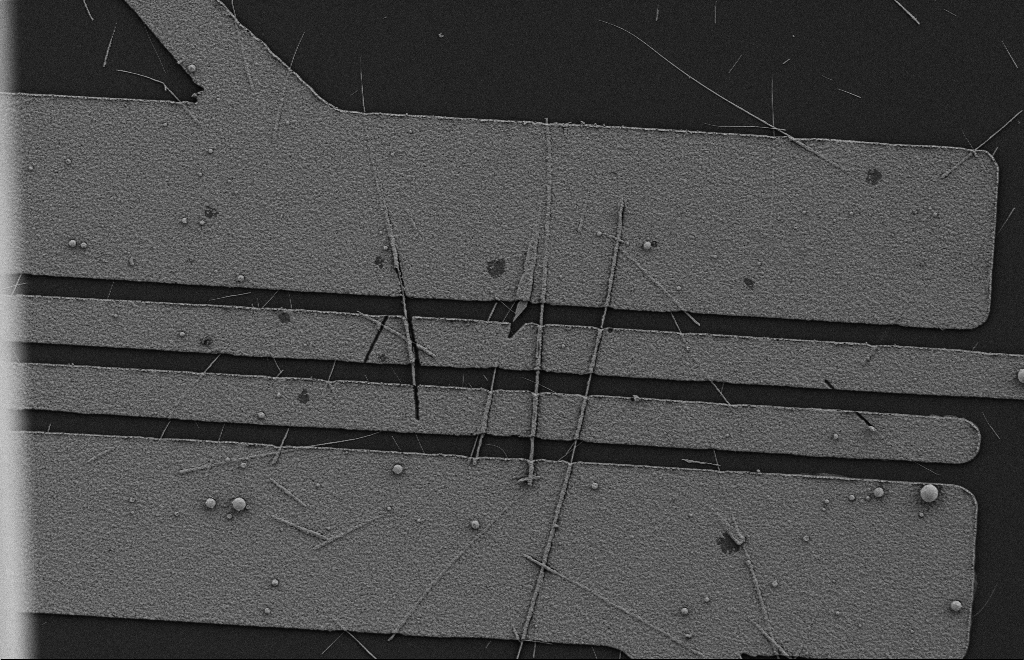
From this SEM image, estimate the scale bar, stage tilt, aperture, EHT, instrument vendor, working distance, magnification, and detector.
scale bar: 2000 nm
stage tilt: -0.3°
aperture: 20 µm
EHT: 2 kV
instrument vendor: Zeiss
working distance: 10 mm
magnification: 6.18 K X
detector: SE2